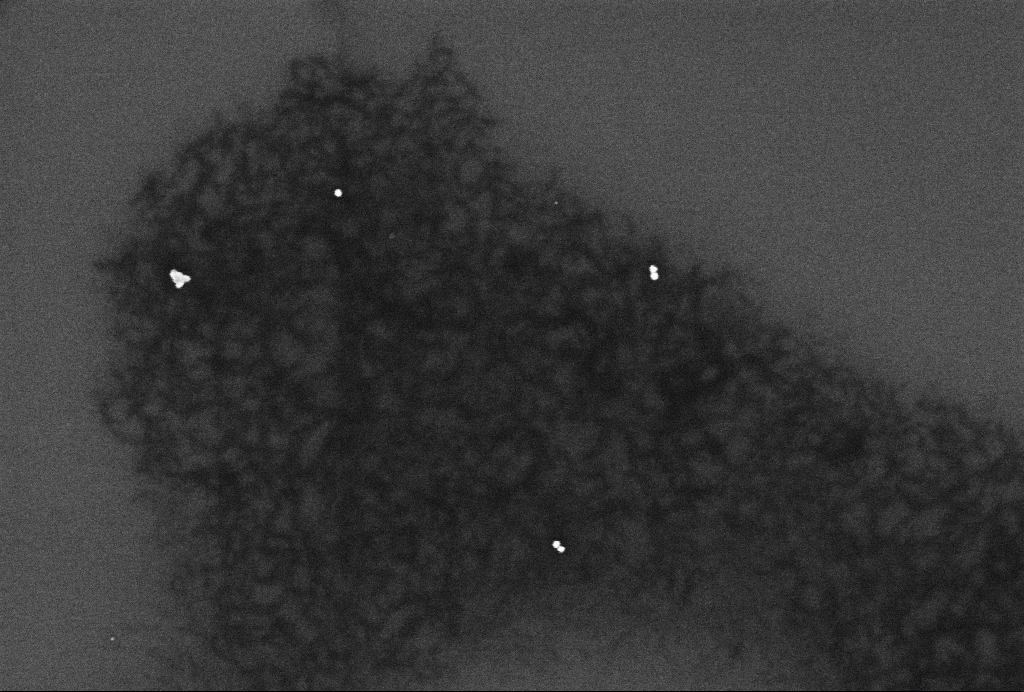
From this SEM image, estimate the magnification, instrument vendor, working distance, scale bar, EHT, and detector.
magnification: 110.36 K X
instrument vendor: Zeiss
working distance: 3.3 mm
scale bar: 200 nm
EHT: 2 kV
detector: InLens